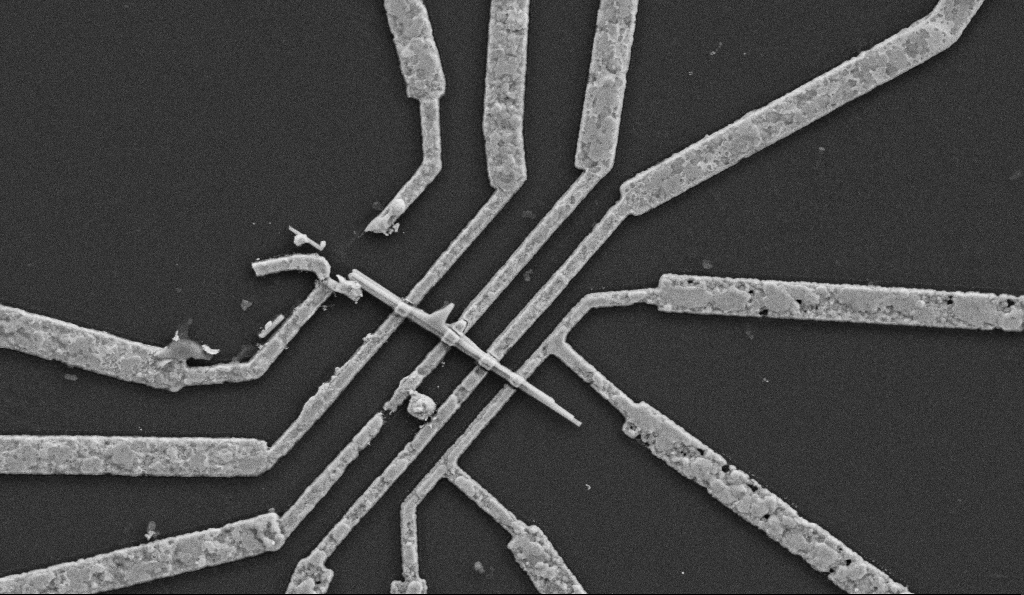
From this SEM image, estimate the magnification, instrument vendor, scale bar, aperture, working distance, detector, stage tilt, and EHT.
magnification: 20 K X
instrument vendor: Zeiss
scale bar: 1000 nm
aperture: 30 µm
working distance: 8.5 mm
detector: SE2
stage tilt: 0°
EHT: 5 kV